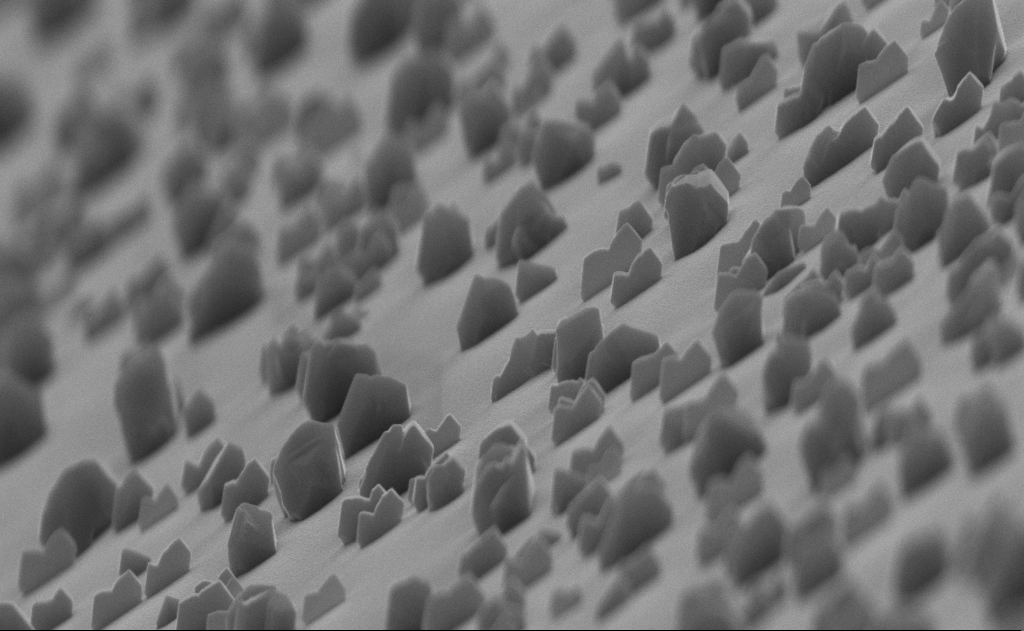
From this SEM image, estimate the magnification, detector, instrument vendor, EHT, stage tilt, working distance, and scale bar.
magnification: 40 K X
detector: InLens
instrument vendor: Zeiss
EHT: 10 kV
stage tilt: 0°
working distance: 12 mm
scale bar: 1000 nm